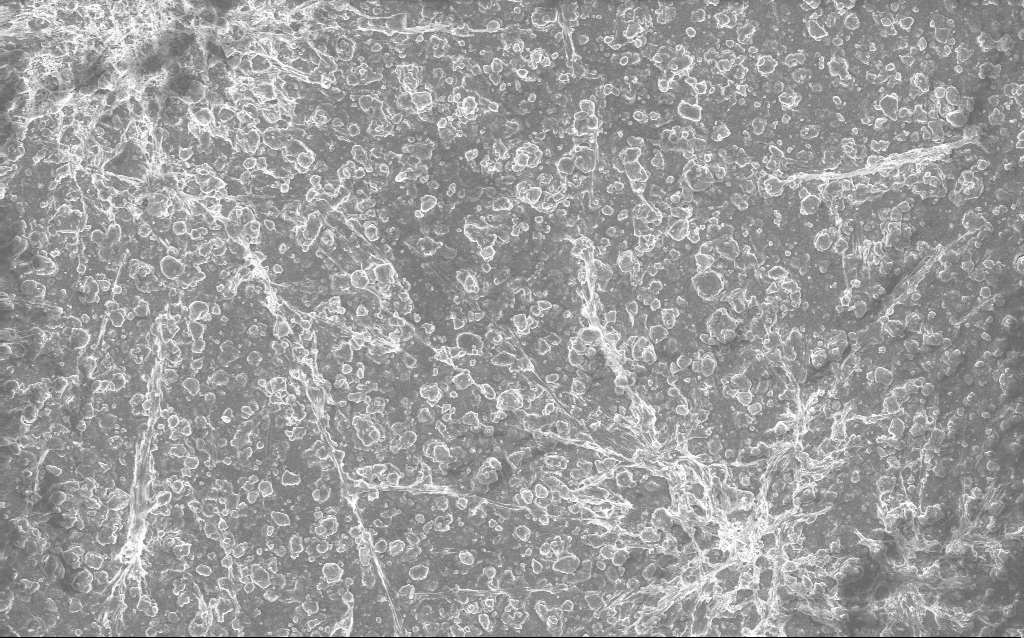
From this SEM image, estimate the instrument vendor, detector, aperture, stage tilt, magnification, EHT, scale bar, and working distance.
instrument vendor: Zeiss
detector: InLens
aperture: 30 µm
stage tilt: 0°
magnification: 0.792 K X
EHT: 10 kV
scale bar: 20000 nm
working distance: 2.7 mm